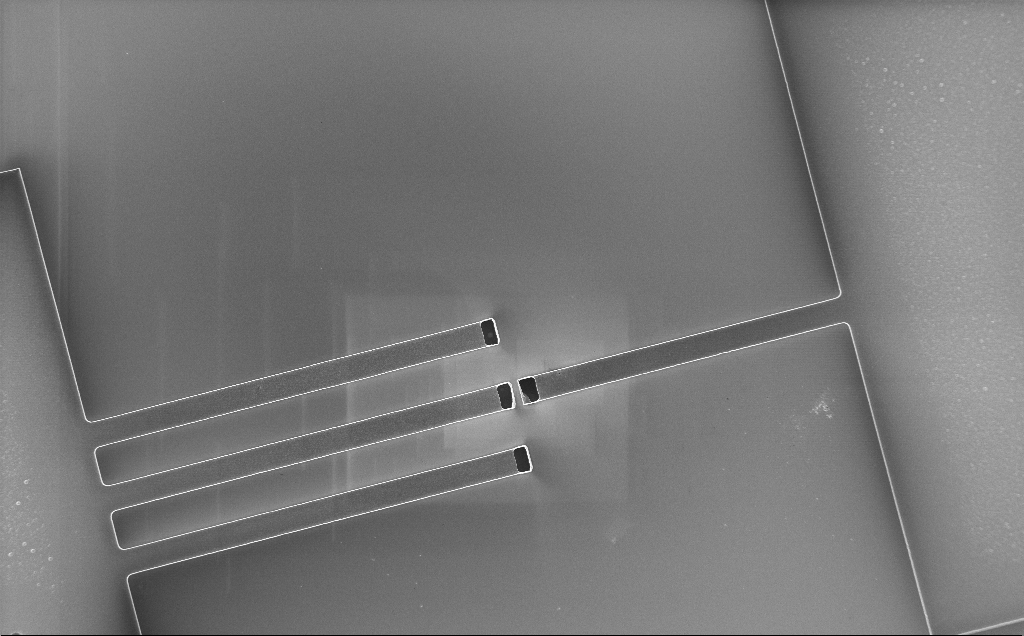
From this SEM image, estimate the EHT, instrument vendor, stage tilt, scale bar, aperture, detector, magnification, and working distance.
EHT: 2 kV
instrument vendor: Zeiss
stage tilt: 0°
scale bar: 100000 nm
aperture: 30 µm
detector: InLens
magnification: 0.604 K X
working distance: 10 mm